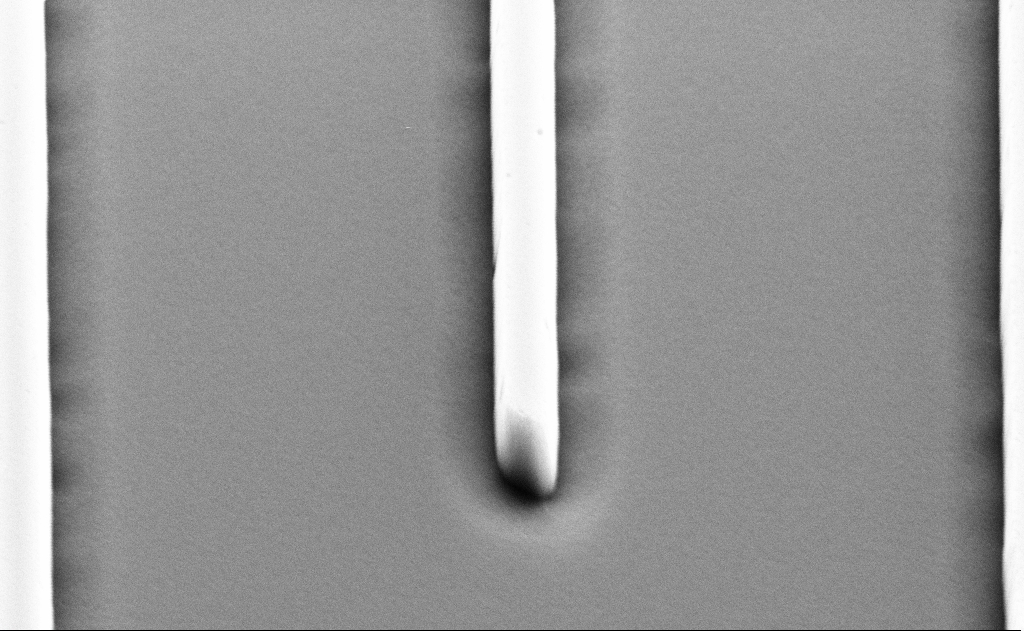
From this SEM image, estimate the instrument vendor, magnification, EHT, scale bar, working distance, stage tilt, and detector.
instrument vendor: Zeiss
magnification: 46.5 K X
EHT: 5 kV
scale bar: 1000 nm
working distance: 11 mm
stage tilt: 45°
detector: SE2